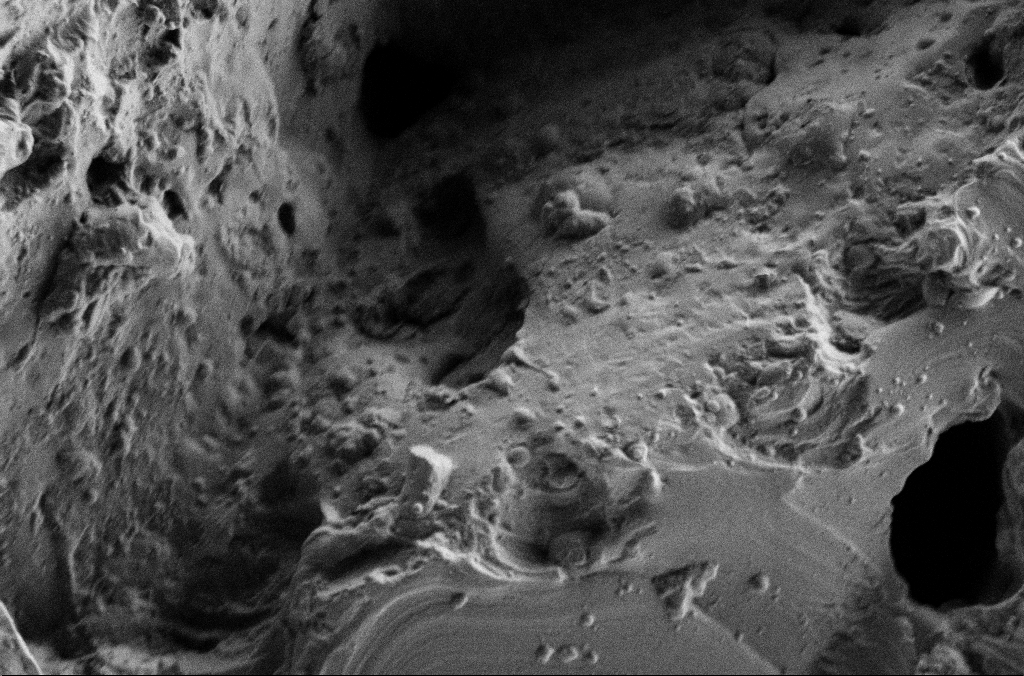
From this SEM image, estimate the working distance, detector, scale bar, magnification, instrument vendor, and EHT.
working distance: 3 mm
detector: SE2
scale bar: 10000 nm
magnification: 5 K X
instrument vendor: Zeiss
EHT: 2 kV